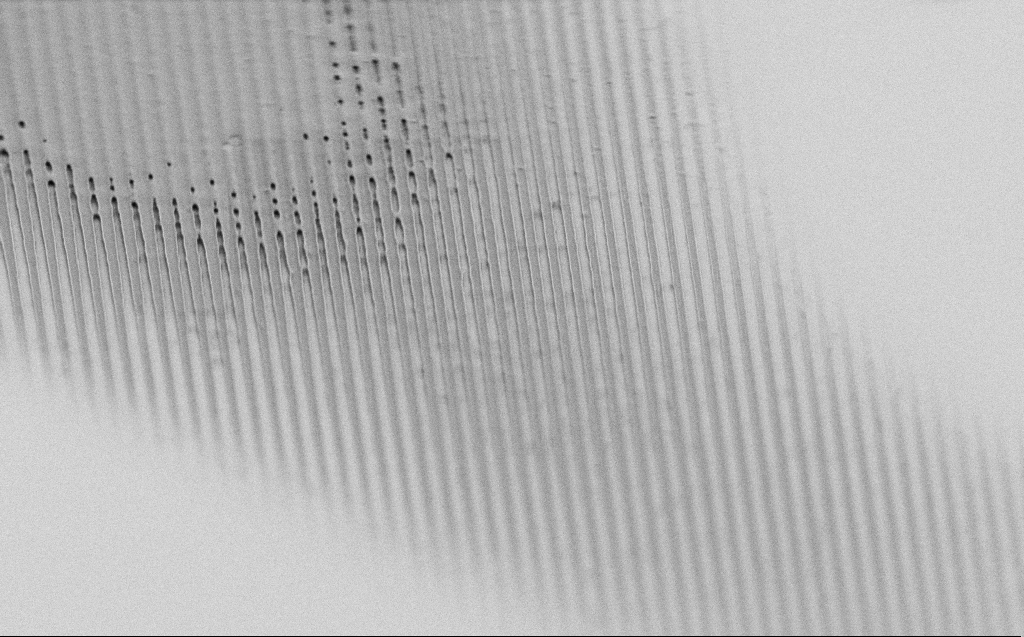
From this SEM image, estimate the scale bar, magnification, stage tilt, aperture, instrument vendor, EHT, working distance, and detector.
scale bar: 2000 nm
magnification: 14.96 K X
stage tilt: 60.7°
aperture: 30 µm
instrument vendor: Zeiss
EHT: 1 kV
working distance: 3 mm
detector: SE2